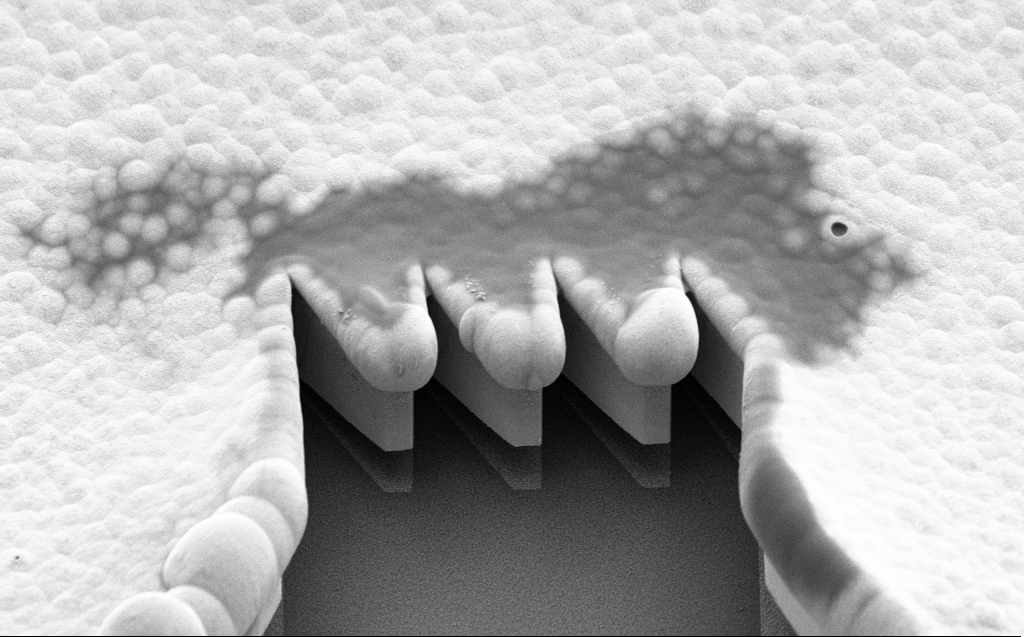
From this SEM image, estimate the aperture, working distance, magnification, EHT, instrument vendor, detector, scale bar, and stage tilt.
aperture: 30 µm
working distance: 9 mm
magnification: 4.15 K X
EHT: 5 kV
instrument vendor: Zeiss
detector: SE2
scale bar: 10000 nm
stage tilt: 45°